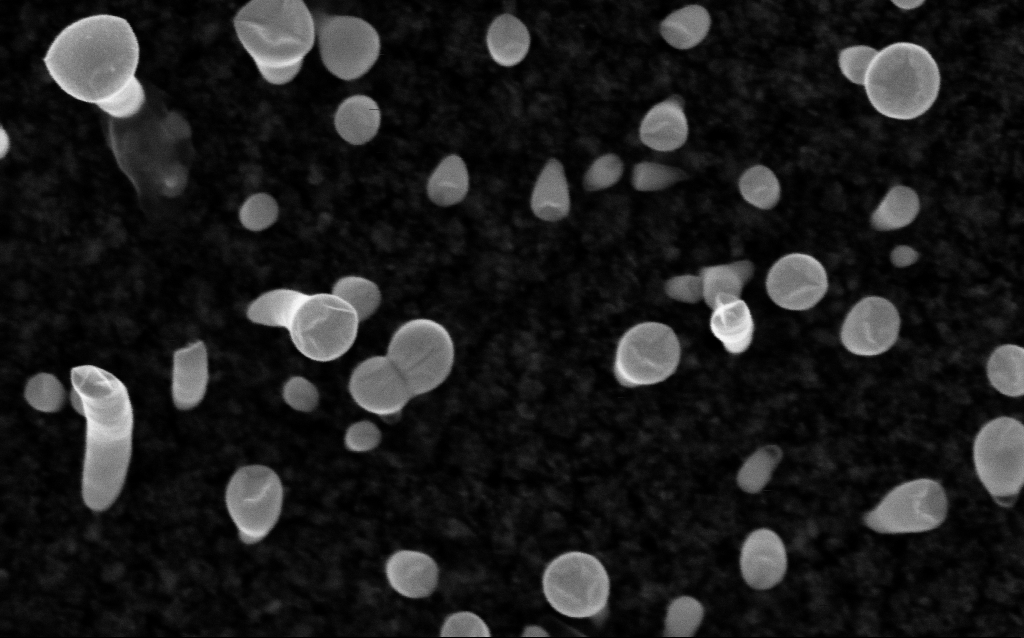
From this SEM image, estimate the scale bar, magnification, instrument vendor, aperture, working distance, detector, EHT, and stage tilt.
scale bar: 100 nm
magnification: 200 K X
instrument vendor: Zeiss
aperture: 30 µm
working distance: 2.1 mm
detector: InLens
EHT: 5 kV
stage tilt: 0°